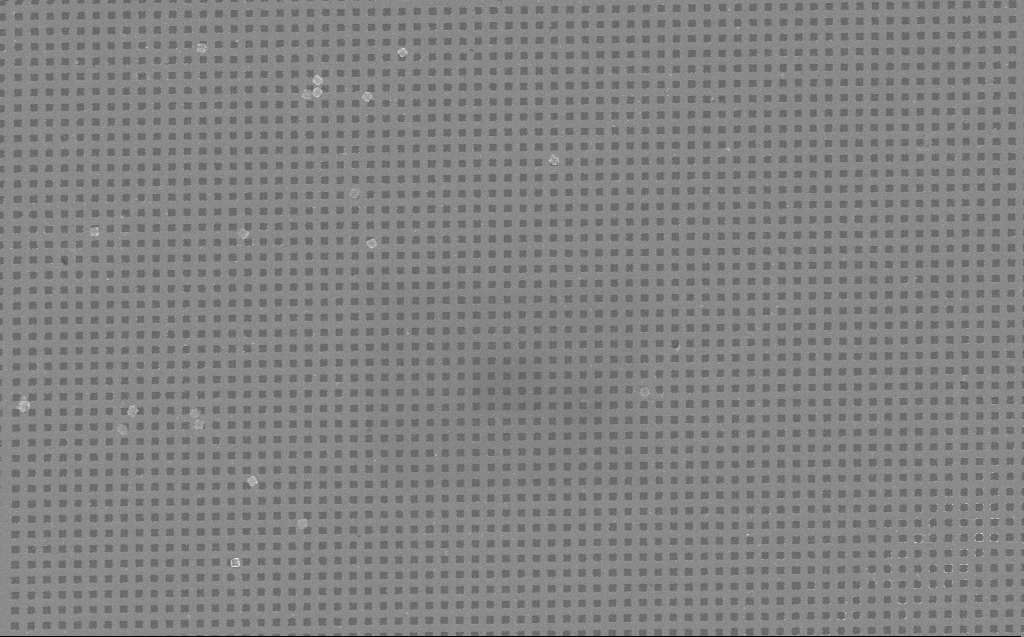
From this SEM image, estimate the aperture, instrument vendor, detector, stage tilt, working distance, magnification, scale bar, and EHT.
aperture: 30 µm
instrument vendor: Zeiss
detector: InLens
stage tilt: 0°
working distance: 5 mm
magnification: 11.34 K X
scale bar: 2000 nm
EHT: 10 kV